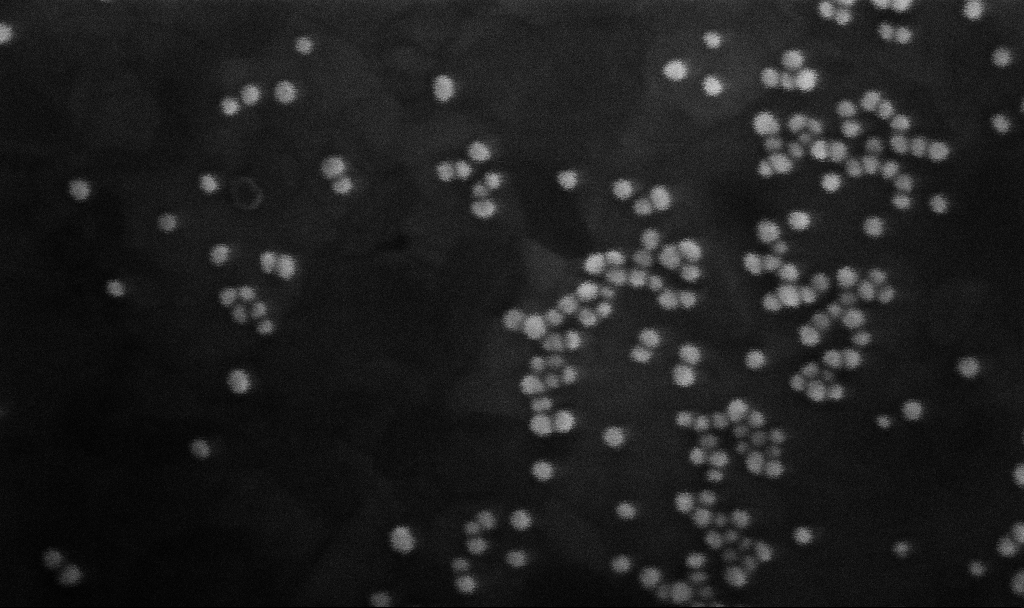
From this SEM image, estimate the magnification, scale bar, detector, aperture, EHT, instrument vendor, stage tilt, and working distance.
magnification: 400 K X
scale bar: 100 nm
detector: InLens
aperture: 30 µm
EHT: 10 kV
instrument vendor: Zeiss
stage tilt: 0°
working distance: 7 mm